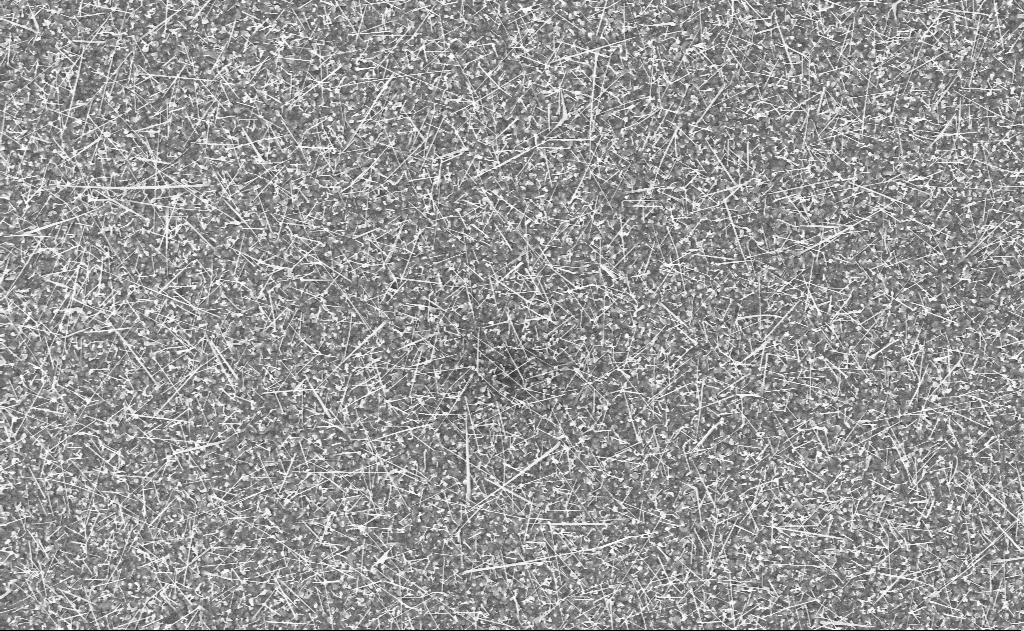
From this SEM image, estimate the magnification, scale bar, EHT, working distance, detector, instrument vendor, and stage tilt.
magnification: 10 K X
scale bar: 2000 nm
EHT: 10 kV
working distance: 20 mm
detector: InLens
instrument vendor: Zeiss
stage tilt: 0°